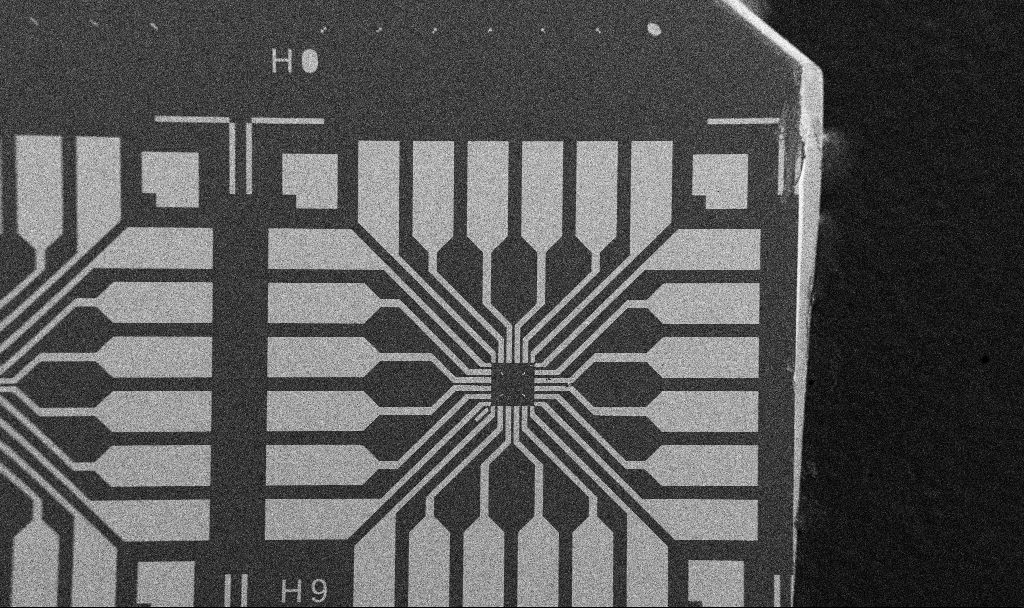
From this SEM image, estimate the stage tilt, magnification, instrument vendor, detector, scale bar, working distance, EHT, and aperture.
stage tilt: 0°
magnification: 0.1 K X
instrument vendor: Zeiss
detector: SE2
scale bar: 200000 nm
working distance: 10.7 mm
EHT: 5 kV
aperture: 30 µm